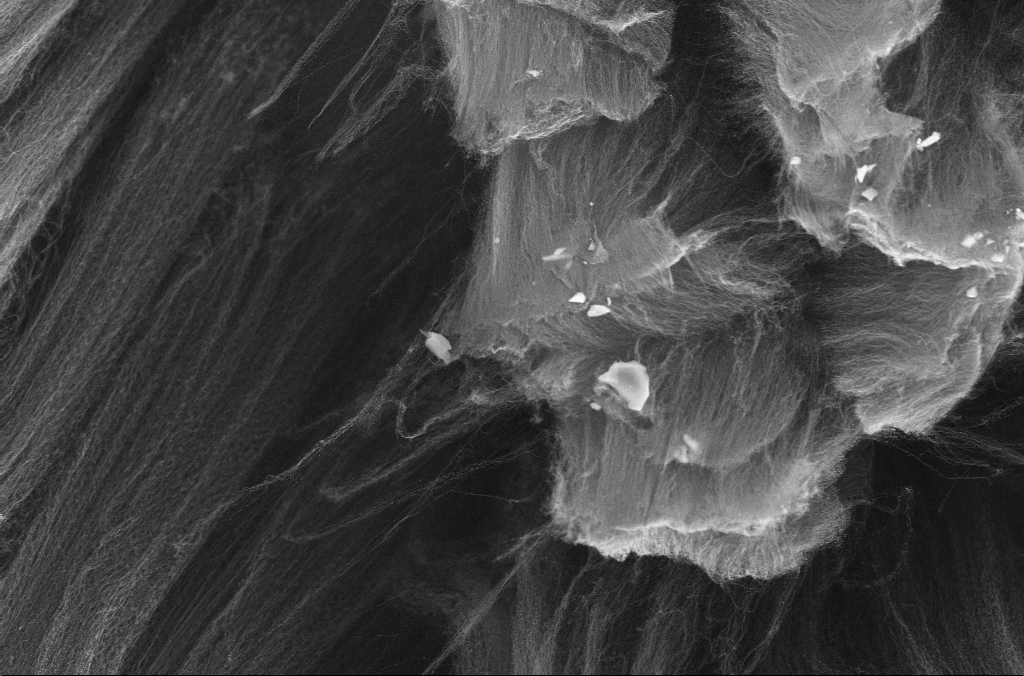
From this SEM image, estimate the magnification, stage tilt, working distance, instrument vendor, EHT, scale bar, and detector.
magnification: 5 K X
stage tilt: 0°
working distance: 4 mm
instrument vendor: Zeiss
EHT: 10 kV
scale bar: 10000 nm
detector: InLens